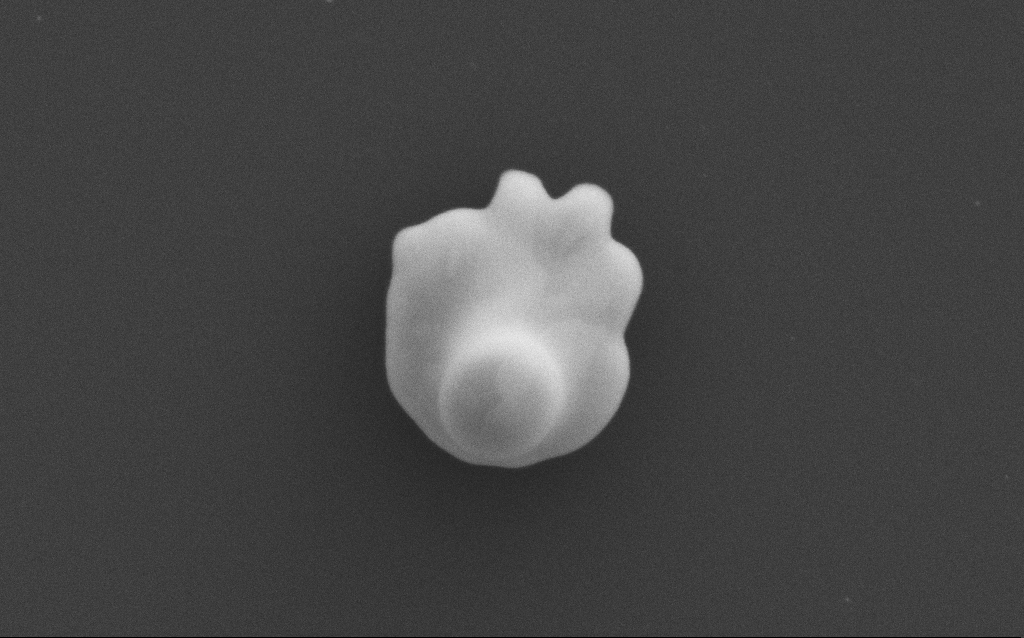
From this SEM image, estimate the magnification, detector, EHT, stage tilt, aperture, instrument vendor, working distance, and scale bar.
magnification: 66.94 K X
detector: SE2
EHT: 10 kV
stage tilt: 0°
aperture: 30 µm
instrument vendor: Zeiss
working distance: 3 mm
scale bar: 1000 nm